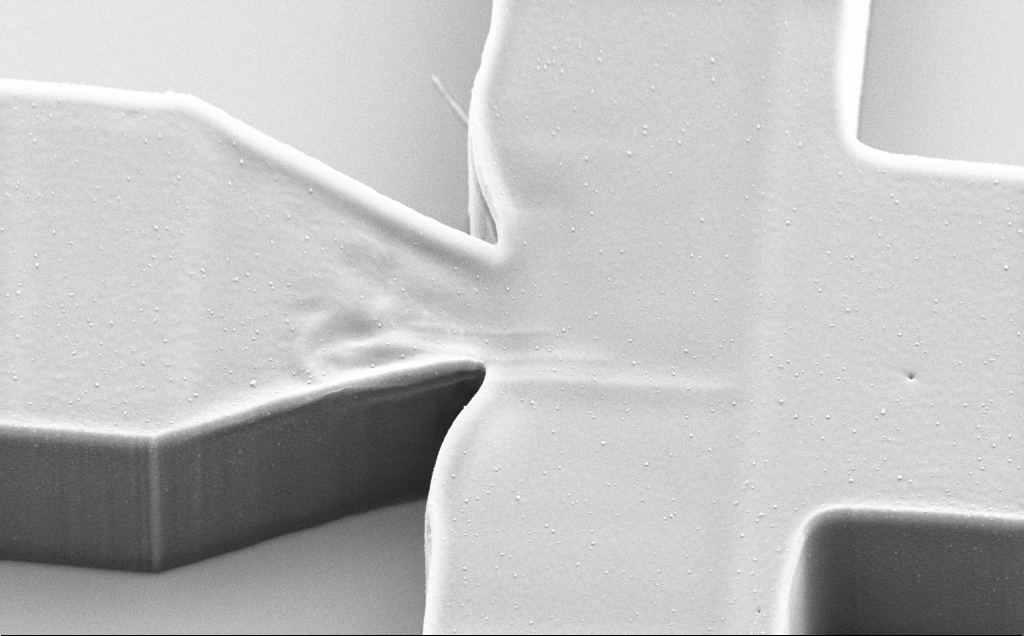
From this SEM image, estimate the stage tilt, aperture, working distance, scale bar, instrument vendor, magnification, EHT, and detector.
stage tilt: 45°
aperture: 30 µm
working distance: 9 mm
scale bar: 1000 nm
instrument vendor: Zeiss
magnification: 12.9 K X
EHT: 5 kV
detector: SE2